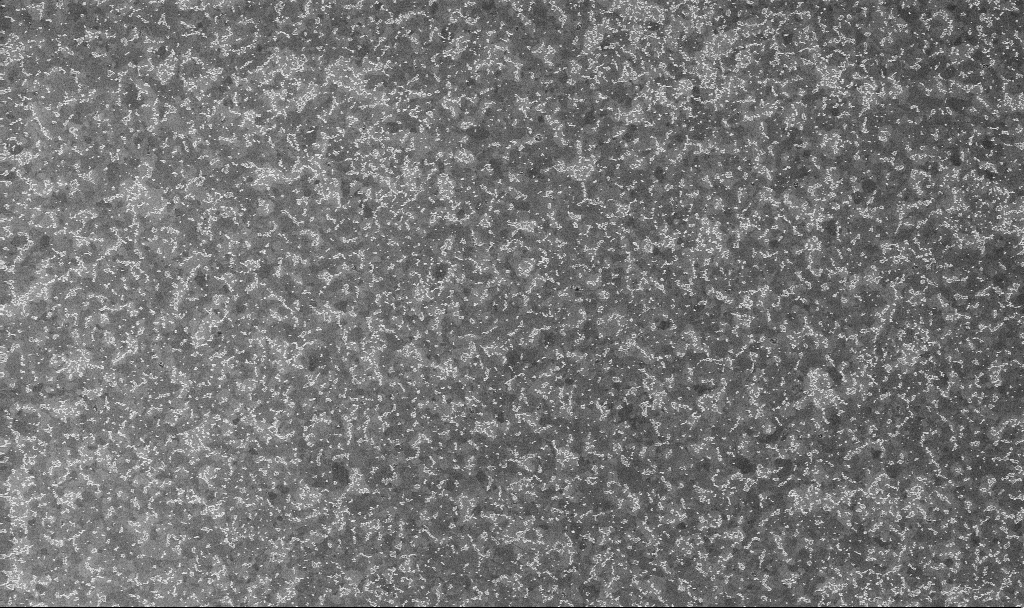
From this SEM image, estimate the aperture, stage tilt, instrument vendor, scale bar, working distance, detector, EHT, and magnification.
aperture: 30 µm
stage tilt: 0°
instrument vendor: Zeiss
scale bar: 1000 nm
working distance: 3.3 mm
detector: InLens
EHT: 10 kV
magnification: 20 K X